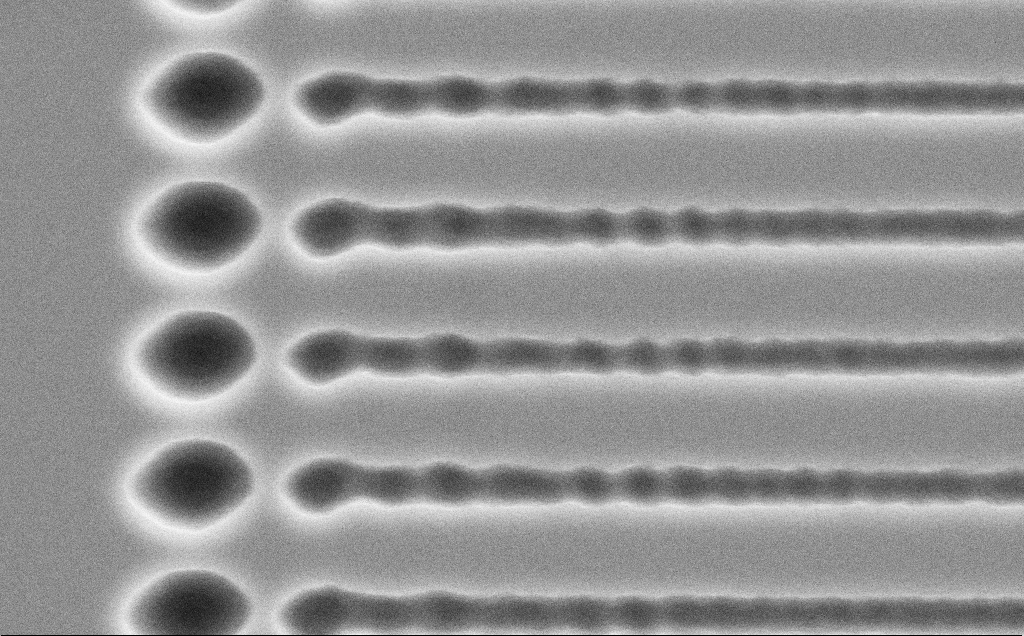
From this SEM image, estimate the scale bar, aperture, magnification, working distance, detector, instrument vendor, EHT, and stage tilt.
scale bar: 2000 nm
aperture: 30 µm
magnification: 24.07 K X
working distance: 10 mm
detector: SE2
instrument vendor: Zeiss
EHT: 5 kV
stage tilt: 0°